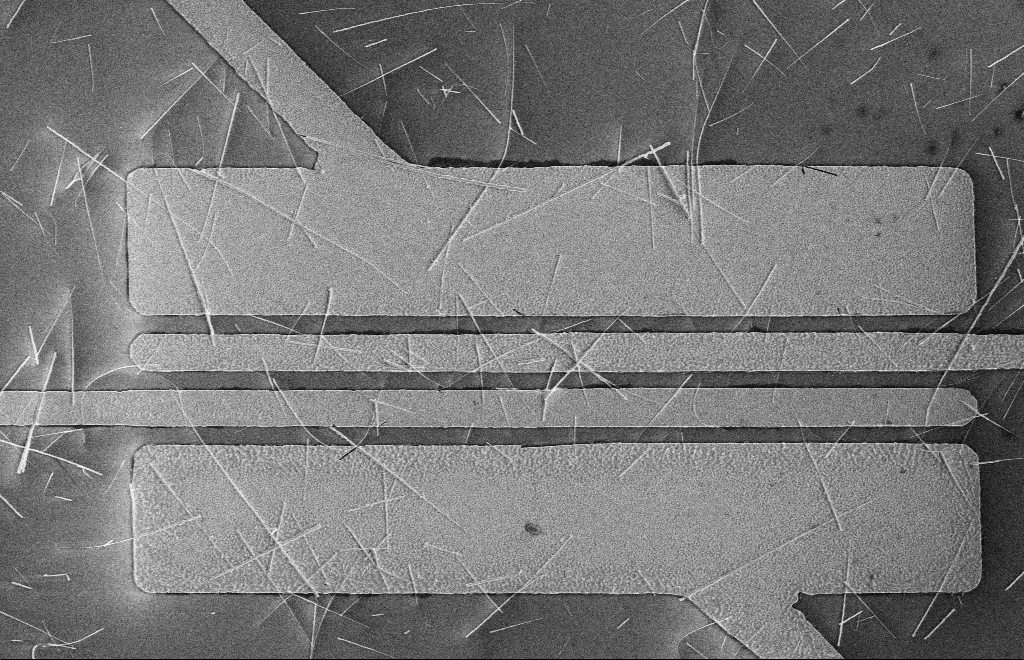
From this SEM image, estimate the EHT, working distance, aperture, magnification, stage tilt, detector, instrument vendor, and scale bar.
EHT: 5 kV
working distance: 16 mm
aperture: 10 µm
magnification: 5.13 K X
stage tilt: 0°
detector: SE2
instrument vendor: Zeiss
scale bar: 2000 nm